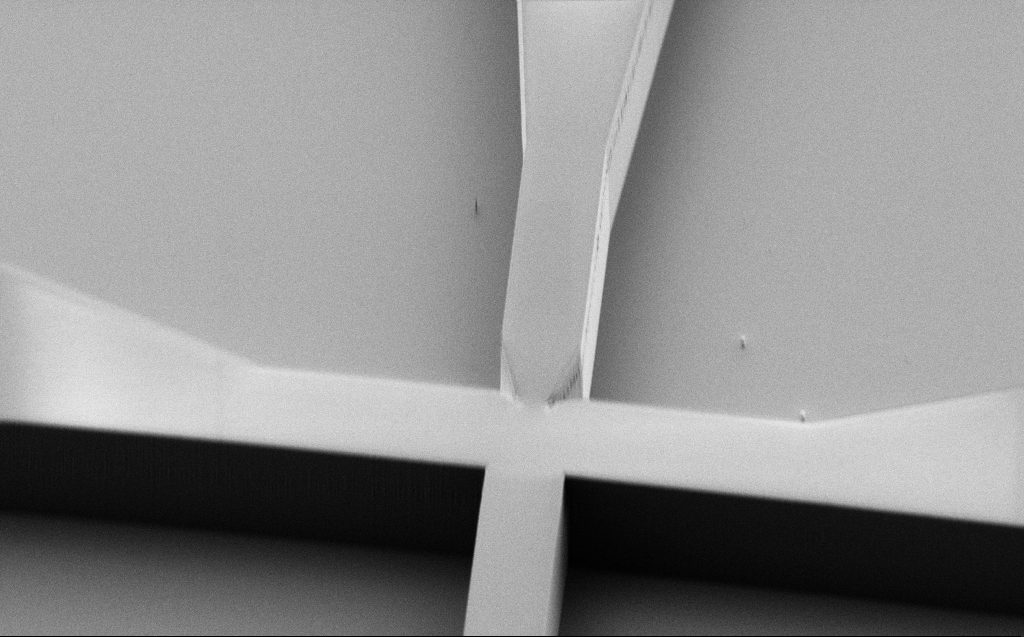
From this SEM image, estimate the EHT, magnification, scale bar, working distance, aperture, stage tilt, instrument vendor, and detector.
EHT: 1.1 kV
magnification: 2.53 K X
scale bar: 20000 nm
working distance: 7 mm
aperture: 30 µm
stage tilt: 45°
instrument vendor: Zeiss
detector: SE2